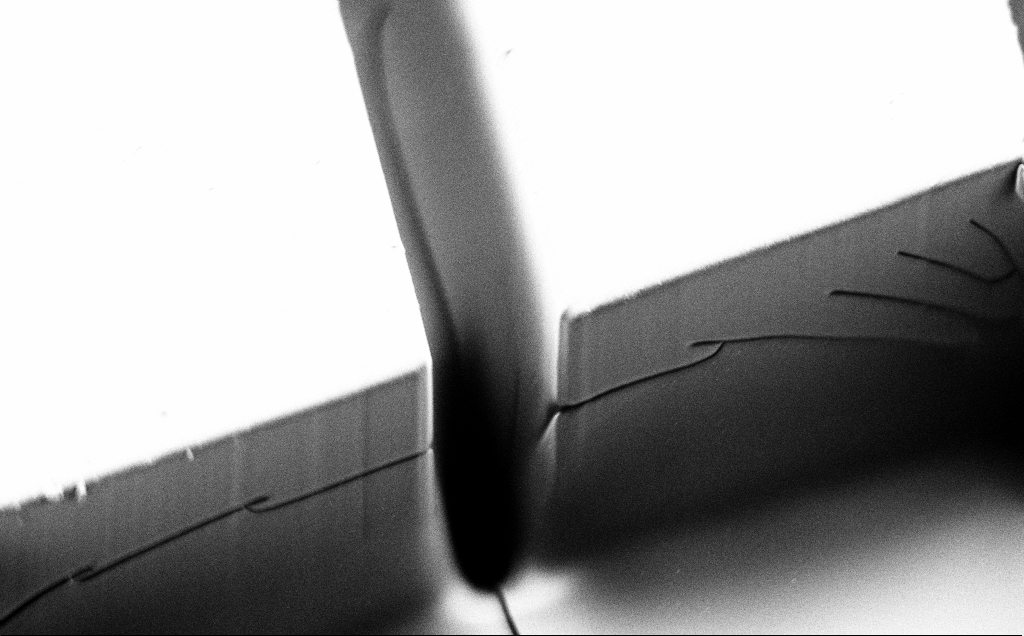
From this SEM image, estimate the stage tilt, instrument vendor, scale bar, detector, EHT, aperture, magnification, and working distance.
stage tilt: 39.3°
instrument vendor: Zeiss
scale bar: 10000 nm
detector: SE2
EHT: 1.2 kV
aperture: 120 µm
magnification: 2.15 K X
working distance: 4 mm